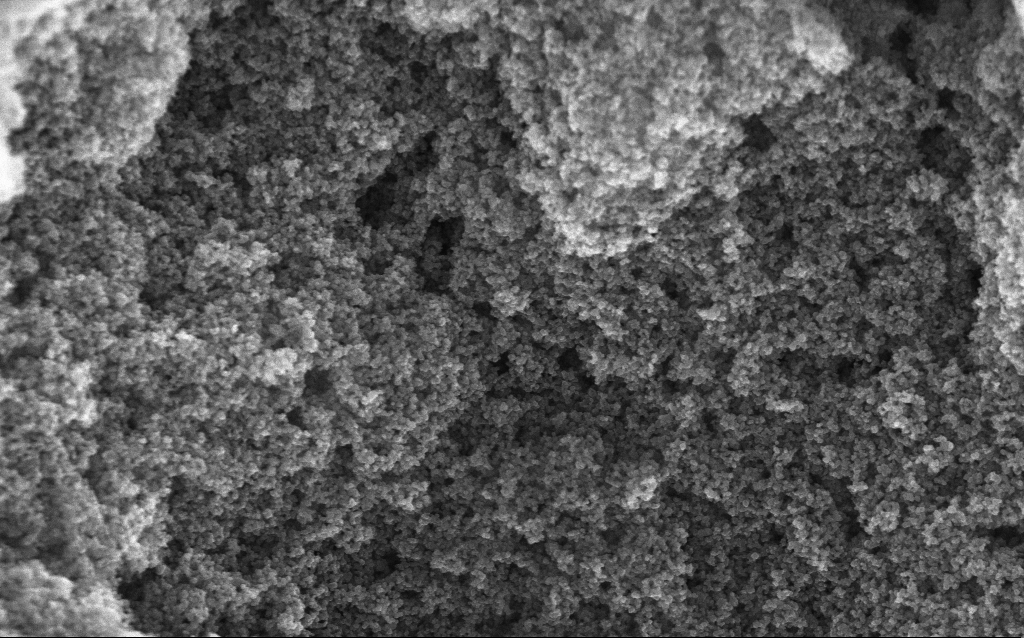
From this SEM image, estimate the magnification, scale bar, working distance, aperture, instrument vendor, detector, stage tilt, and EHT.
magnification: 65.04 K X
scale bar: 1000 nm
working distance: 2.8 mm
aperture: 30 µm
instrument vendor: Zeiss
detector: InLens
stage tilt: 0°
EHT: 10 kV